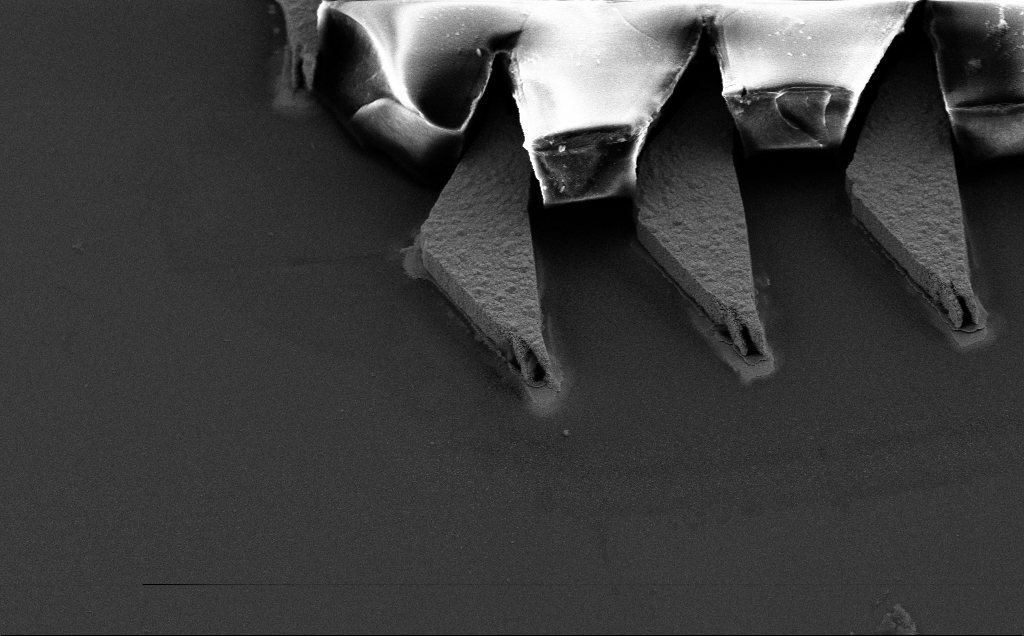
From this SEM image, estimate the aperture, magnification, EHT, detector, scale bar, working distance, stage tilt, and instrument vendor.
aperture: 30 µm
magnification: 3.32 K X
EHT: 10 kV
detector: SE2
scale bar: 20000 nm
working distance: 8 mm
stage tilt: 35°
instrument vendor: Zeiss